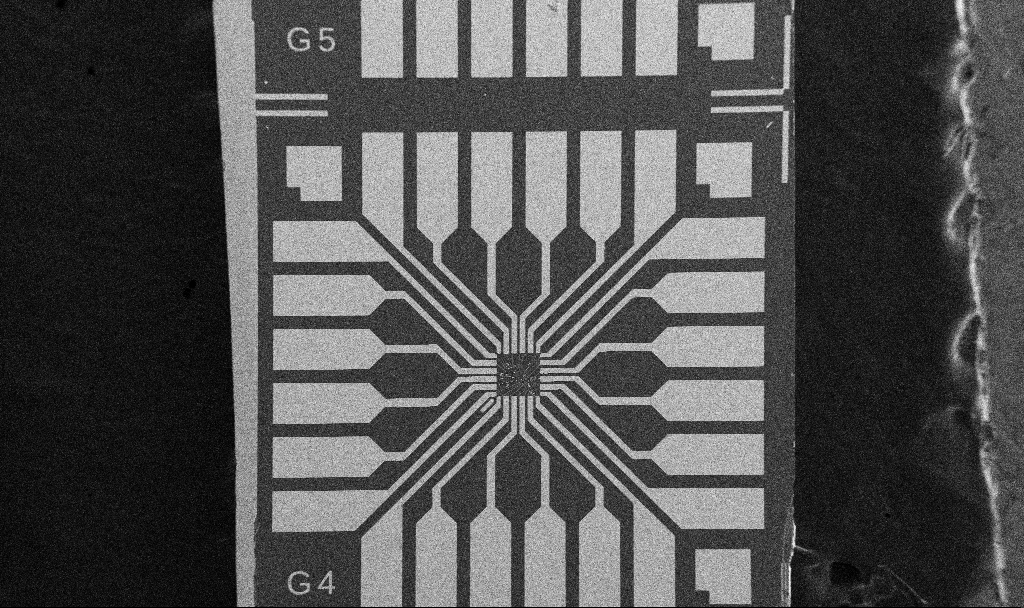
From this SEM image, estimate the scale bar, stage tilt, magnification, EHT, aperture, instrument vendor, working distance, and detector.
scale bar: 200000 nm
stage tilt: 0°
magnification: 0.1 K X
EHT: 5 kV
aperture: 30 µm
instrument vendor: Zeiss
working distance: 10.7 mm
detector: SE2